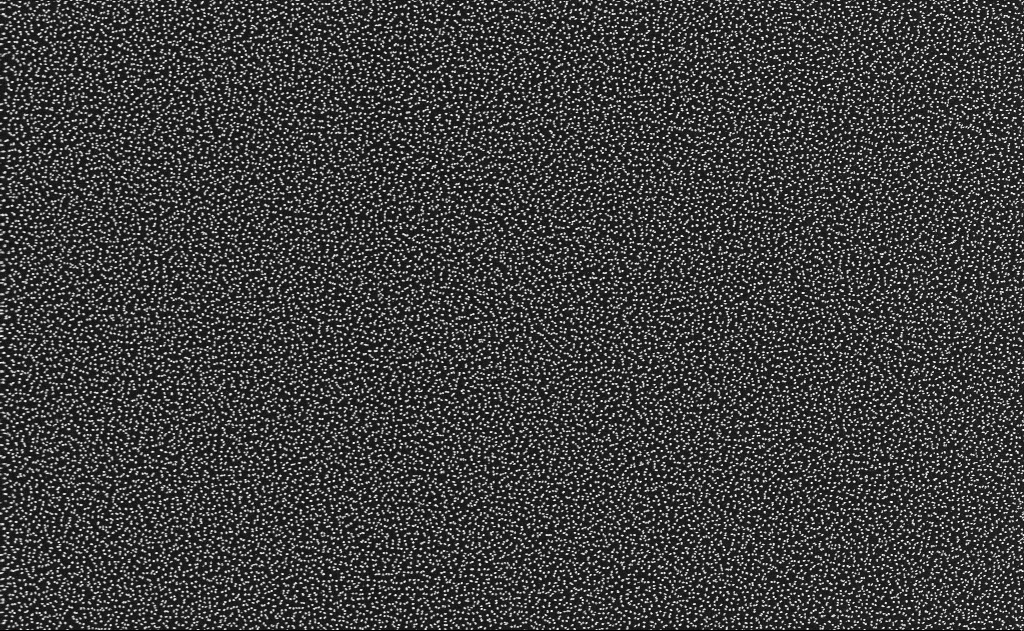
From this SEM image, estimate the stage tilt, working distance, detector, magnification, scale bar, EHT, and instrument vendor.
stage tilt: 0°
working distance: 11 mm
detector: InLens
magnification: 10 K X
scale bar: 2000 nm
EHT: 10 kV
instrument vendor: Zeiss